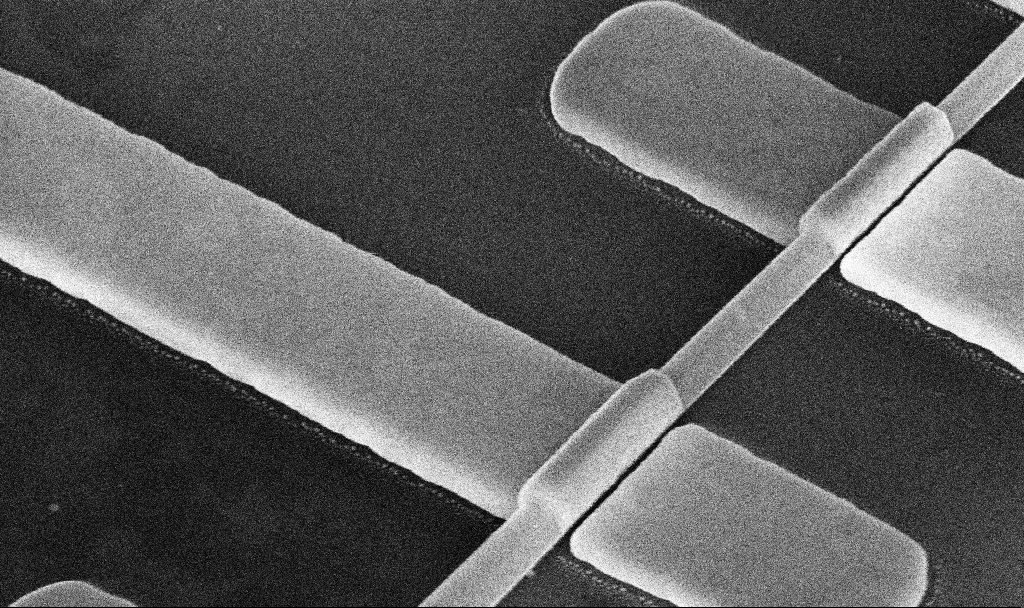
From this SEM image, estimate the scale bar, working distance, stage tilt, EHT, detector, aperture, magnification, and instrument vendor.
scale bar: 200 nm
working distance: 11.1 mm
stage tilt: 45°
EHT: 10 kV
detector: InLens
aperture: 30 µm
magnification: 104.19 K X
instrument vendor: Zeiss